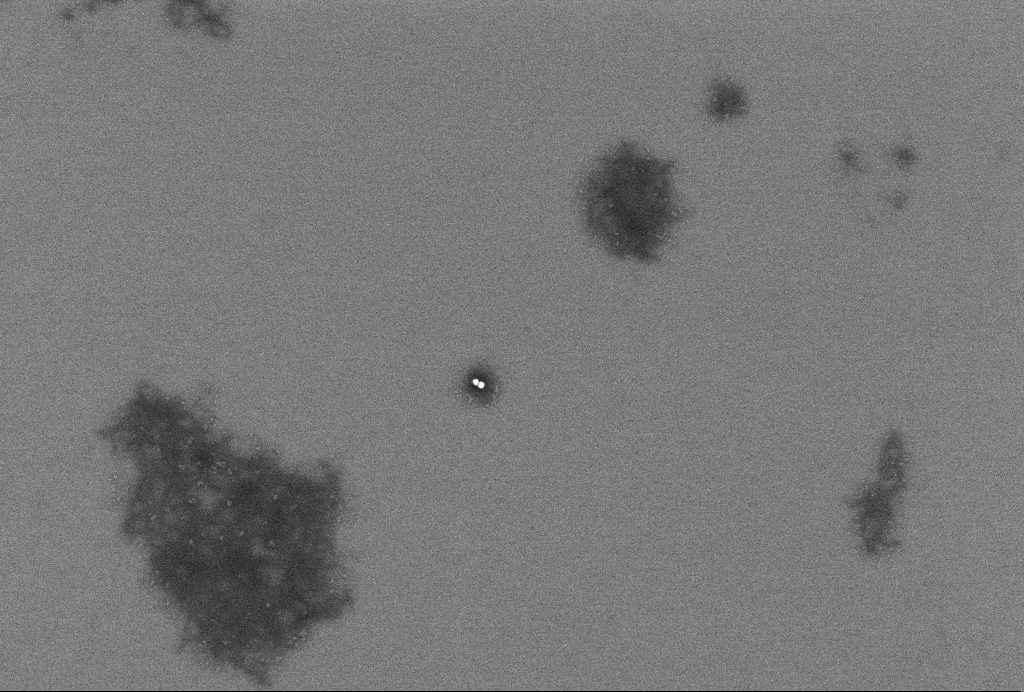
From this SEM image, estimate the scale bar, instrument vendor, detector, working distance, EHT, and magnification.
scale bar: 200 nm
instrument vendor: Zeiss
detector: InLens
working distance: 3.3 mm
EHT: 2 kV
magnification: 86.19 K X